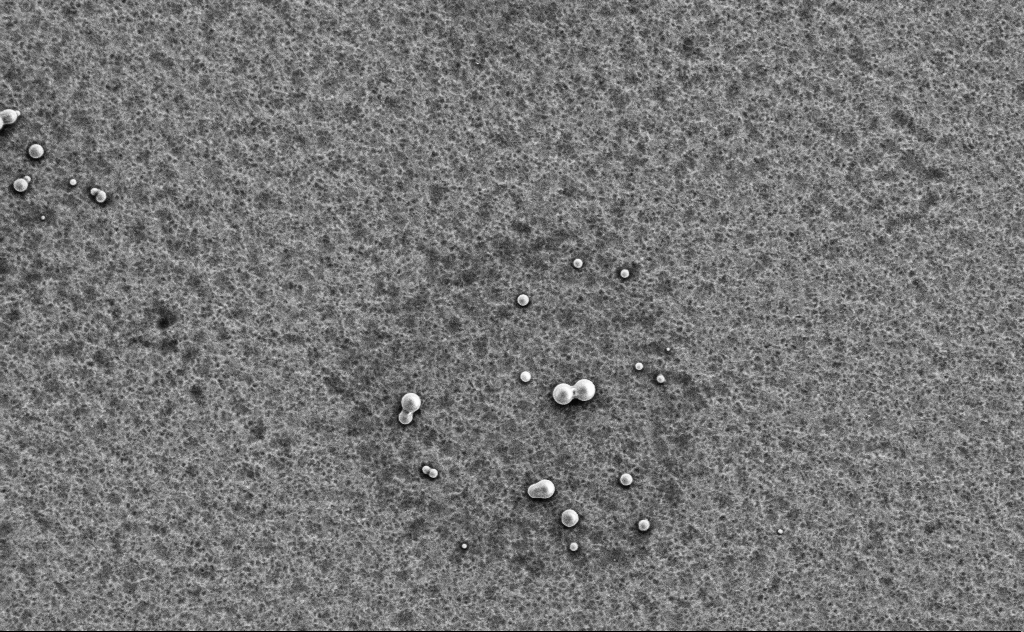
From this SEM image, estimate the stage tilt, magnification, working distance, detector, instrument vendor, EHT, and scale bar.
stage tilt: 0°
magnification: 6.97 K X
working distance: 13 mm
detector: SE2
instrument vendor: Zeiss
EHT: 3 kV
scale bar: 10000 nm